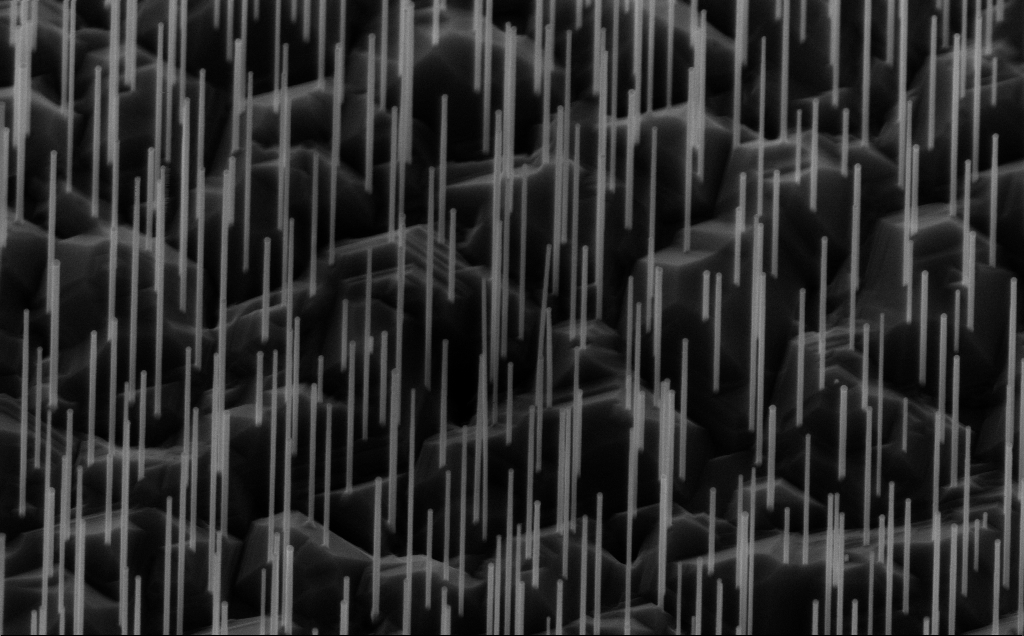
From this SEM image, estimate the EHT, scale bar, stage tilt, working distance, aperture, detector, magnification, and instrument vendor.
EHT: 10 kV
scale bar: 1000 nm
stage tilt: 45°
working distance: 6 mm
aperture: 30 µm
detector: InLens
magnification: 40 K X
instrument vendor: Zeiss